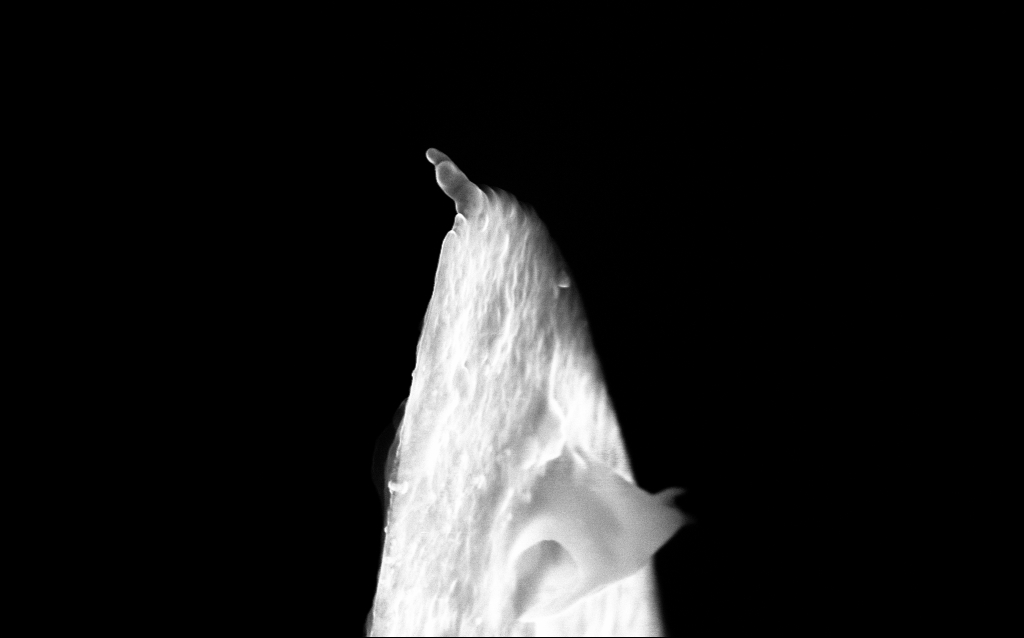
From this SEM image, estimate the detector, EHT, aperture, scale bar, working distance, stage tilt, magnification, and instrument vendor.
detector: InLens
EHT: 10 kV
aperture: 30 µm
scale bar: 200 nm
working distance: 3.7 mm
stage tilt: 45°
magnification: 75.14 K X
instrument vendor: Zeiss